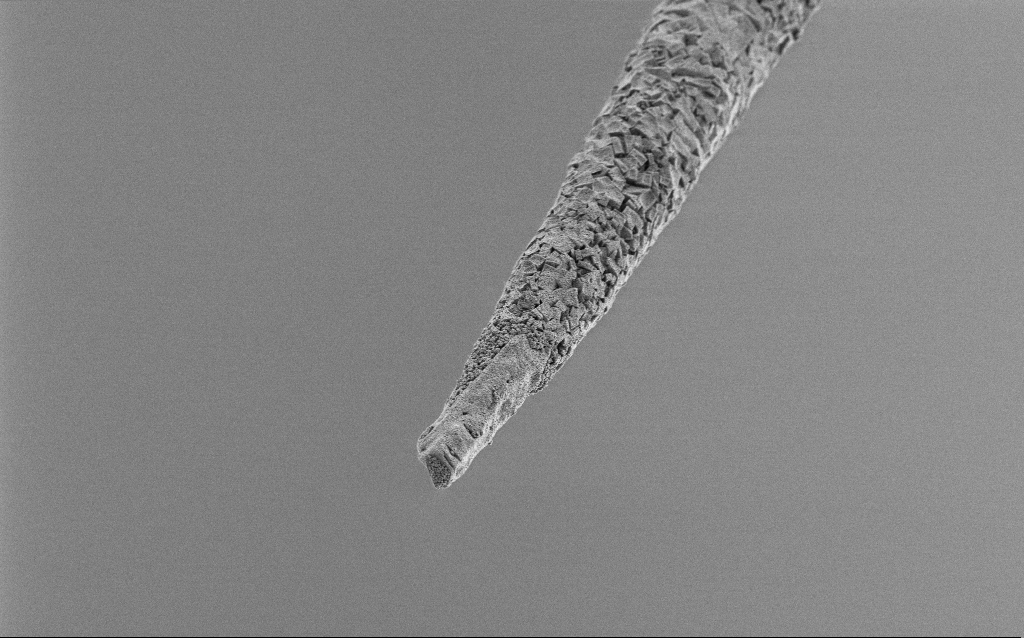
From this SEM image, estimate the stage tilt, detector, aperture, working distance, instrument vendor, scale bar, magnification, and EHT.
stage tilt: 45°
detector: SE2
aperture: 30 µm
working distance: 6.8 mm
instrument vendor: Zeiss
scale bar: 10000 nm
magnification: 2.5 K X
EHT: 1 kV